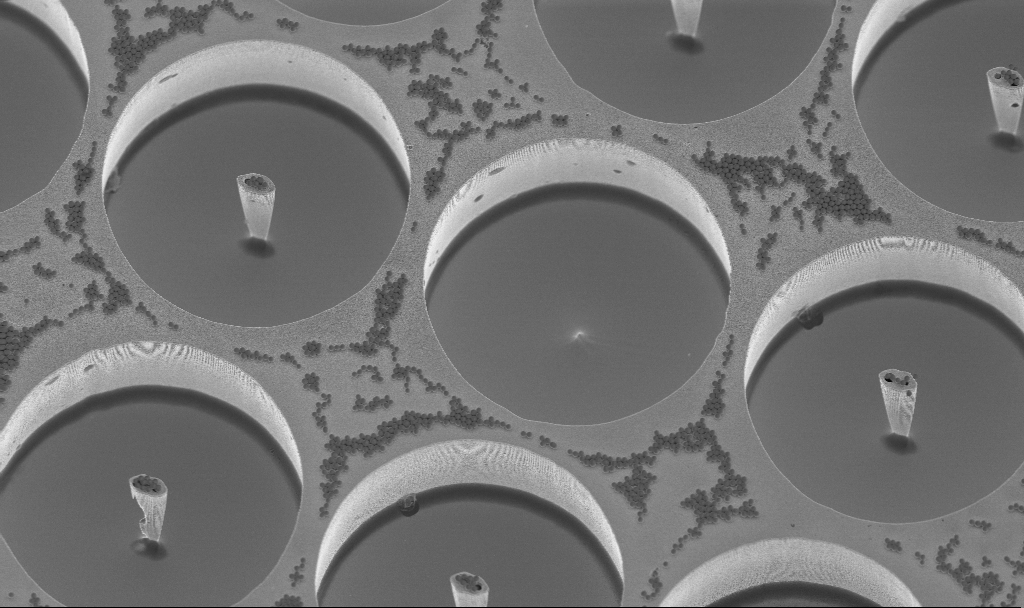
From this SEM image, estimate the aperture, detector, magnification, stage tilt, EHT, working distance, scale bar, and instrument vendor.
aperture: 30 µm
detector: InLens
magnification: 3.78 K X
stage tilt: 20°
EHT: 3 kV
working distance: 3.1 mm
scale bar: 10000 nm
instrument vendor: Zeiss